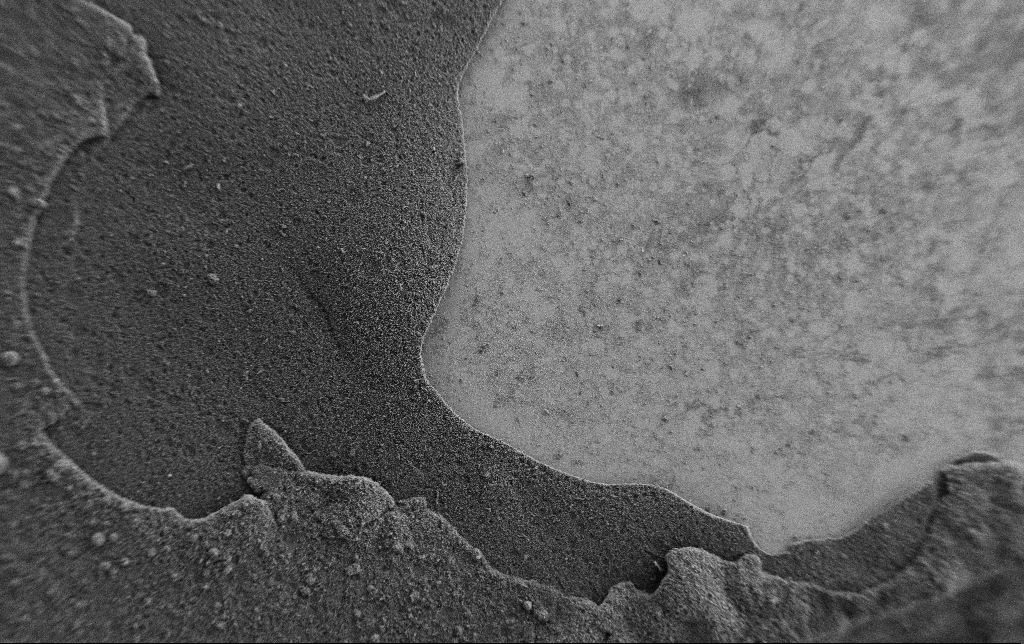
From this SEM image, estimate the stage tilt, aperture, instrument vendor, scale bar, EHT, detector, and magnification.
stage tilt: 0°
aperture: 30 µm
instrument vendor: Zeiss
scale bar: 100000 nm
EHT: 2 kV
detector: SE2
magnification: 0.15 K X